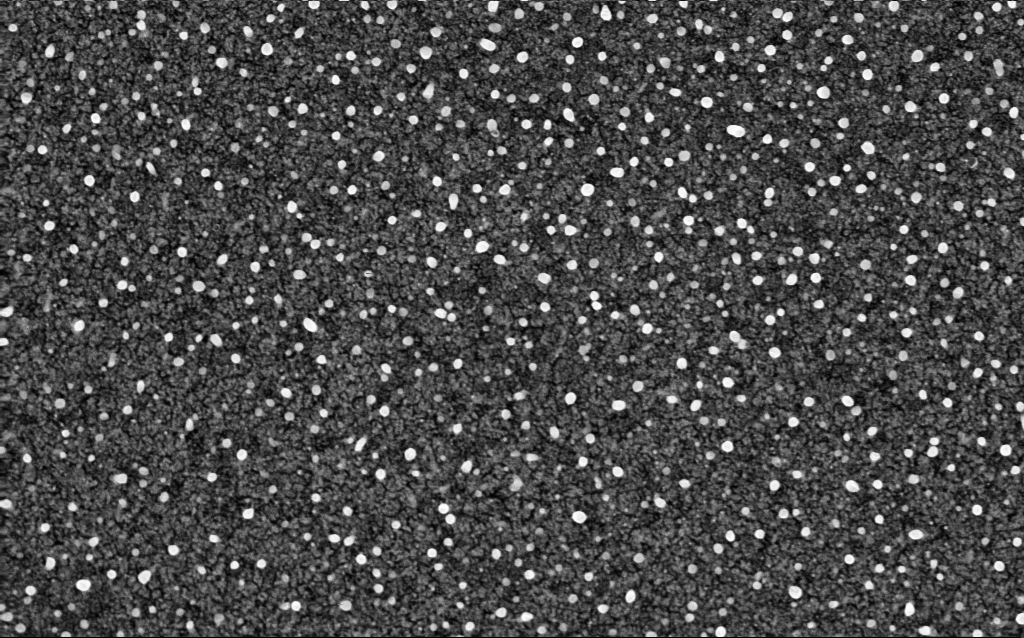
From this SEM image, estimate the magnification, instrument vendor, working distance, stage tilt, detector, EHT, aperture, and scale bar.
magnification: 50 K X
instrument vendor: Zeiss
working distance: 2.1 mm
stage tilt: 0°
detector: InLens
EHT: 5 kV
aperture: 30 µm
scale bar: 1000 nm